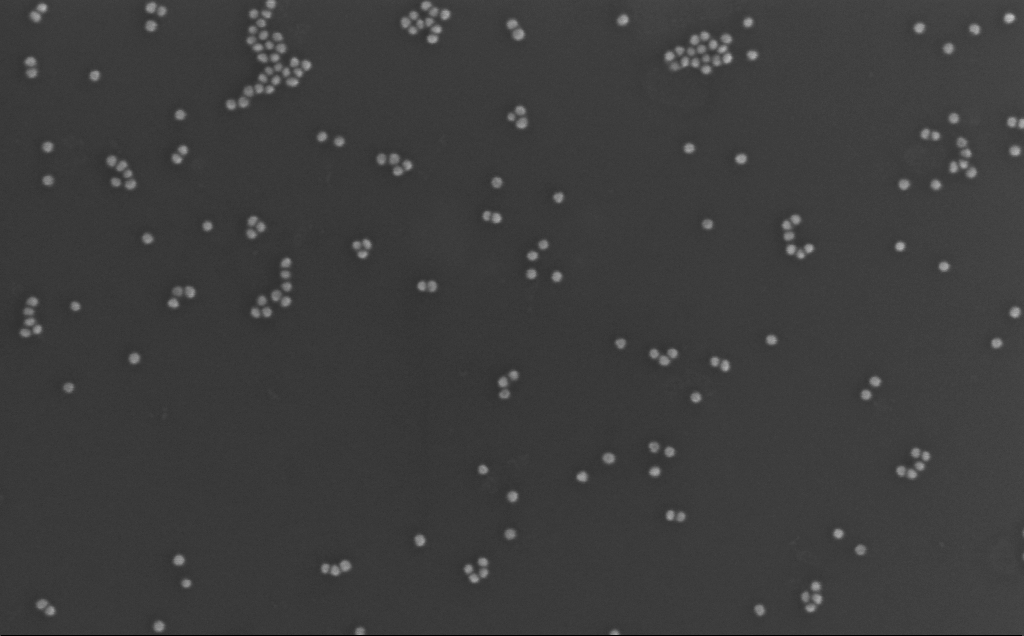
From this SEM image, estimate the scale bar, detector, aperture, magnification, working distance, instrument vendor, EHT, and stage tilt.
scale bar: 200 nm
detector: InLens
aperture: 30 µm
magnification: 223.1 K X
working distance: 3 mm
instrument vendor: Zeiss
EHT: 10 kV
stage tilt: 0°